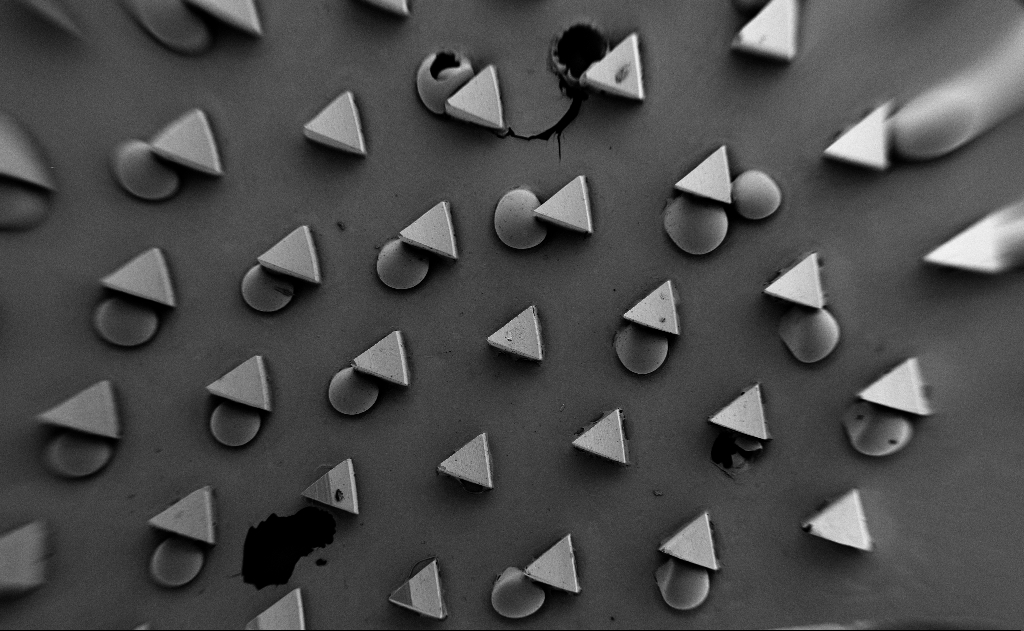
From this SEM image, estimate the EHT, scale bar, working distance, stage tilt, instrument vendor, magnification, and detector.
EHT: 5 kV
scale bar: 1e+06 nm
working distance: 17 mm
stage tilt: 0°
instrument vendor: Zeiss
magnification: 0.052 K X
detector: SE2